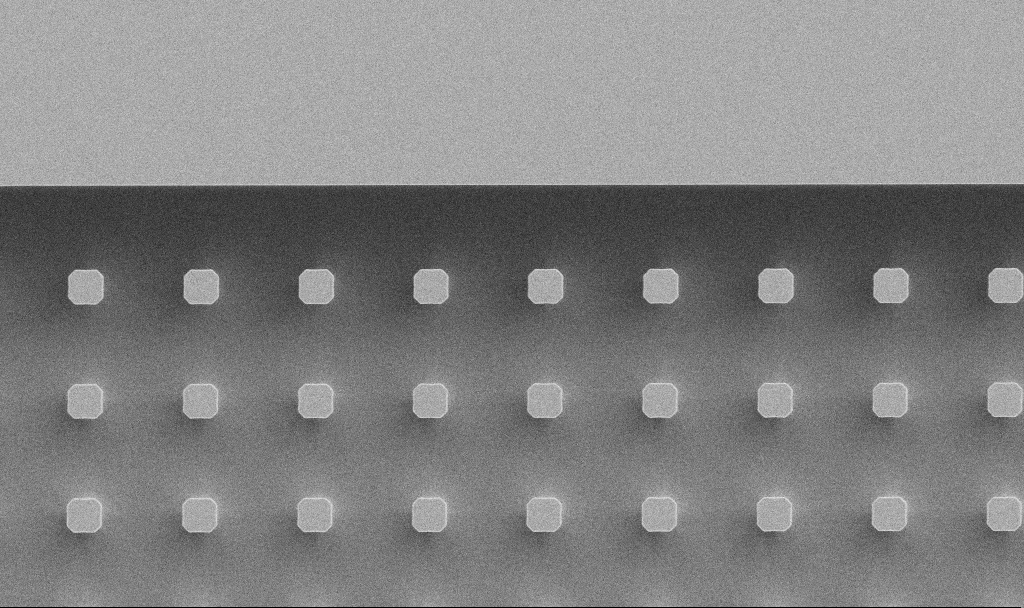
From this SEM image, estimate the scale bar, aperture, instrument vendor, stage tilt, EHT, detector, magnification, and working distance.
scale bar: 20000 nm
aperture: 30 µm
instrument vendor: Zeiss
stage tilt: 0°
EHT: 5 kV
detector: SE2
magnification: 1.41 K X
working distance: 7.7 mm